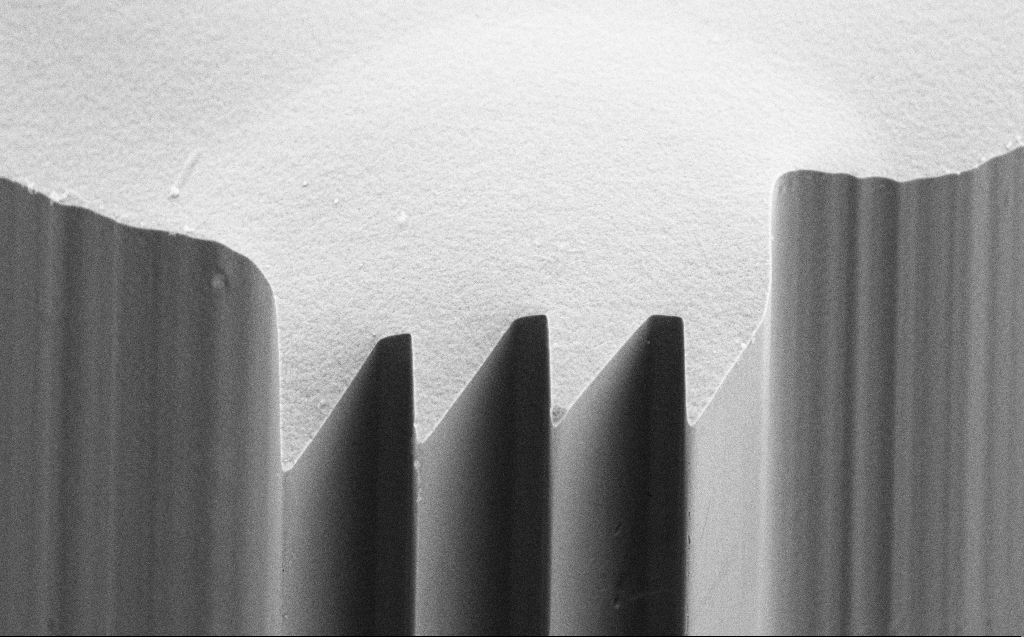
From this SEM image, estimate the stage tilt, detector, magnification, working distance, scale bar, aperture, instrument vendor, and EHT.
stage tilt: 45°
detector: SE2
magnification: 4.37 K X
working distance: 3 mm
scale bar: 10000 nm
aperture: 30 µm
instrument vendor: Zeiss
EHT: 10 kV